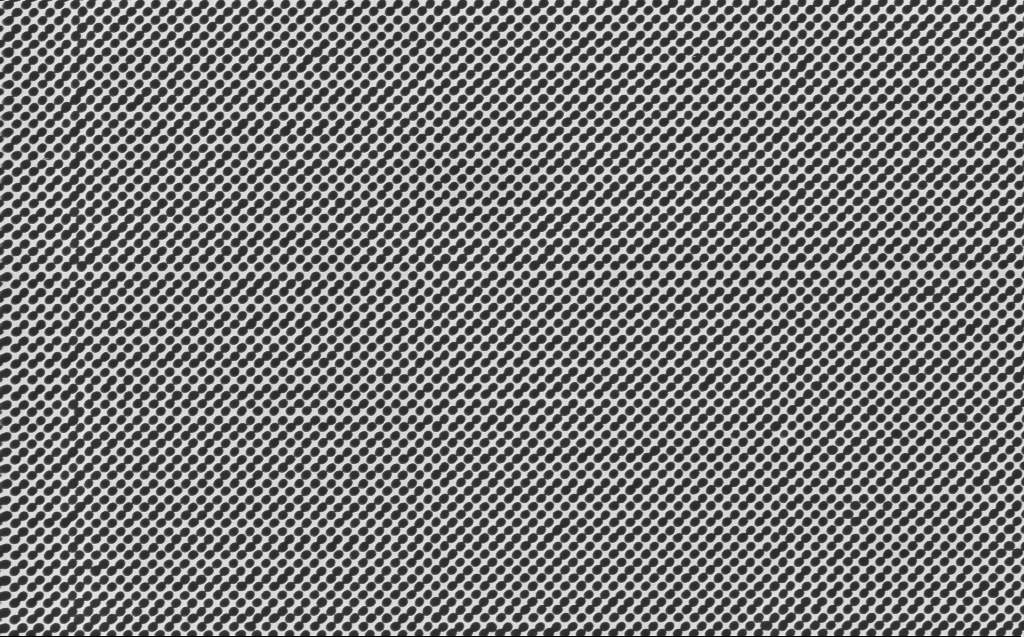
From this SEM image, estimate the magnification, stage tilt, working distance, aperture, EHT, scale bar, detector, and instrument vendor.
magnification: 29.52 K X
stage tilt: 0°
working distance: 7 mm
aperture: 30 µm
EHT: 5 kV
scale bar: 2000 nm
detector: InLens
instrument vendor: Zeiss